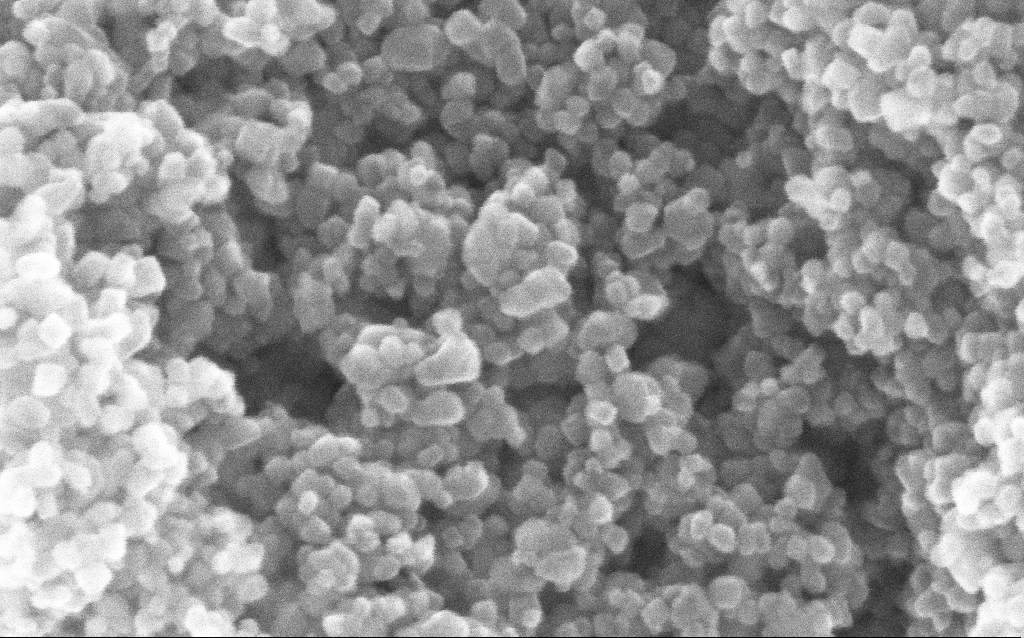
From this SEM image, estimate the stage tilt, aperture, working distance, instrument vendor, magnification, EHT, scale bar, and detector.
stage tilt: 0°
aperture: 30 µm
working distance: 3.8 mm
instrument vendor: Zeiss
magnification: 422.14 K X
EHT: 10 kV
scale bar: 100 nm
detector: InLens